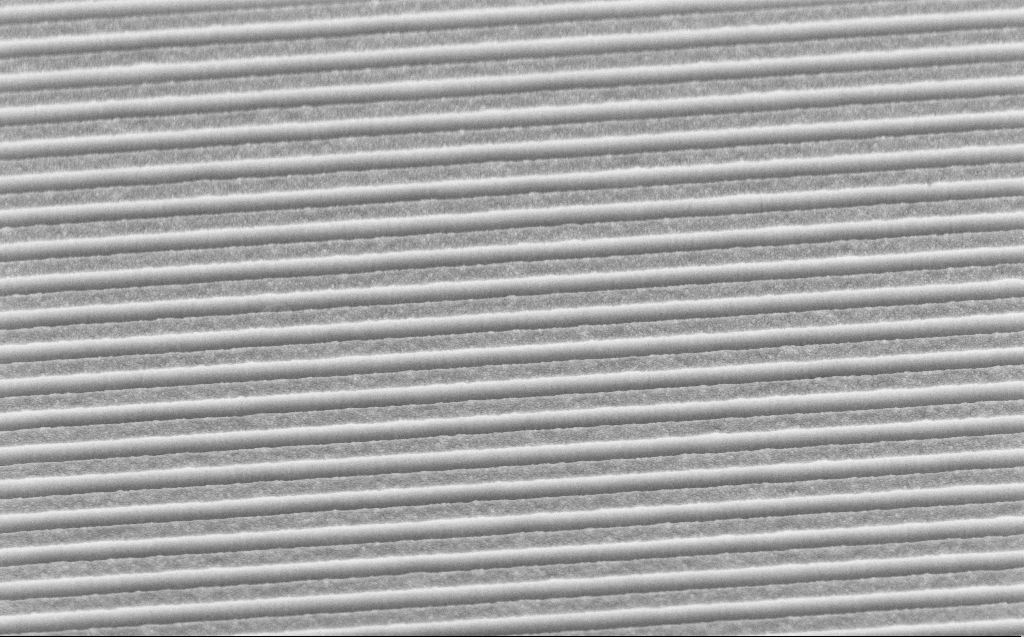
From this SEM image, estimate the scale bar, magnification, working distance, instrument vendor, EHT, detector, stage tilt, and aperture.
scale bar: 2000 nm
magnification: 25.23 K X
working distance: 5 mm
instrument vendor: Zeiss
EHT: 5 kV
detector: InLens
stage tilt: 45°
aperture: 30 µm